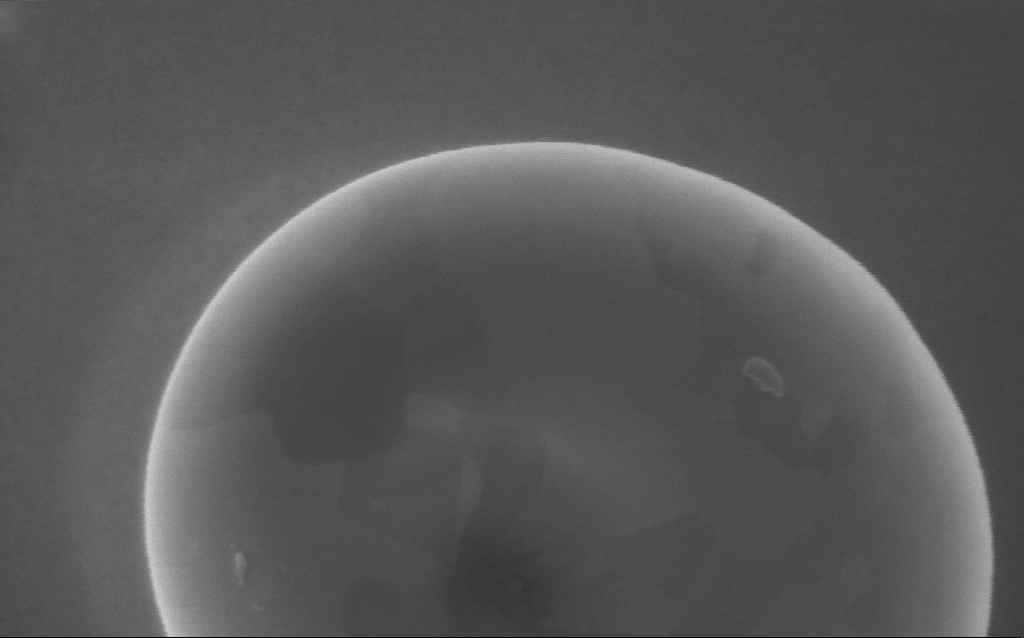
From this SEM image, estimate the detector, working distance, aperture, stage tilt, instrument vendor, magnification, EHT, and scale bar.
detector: InLens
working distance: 4 mm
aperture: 30 µm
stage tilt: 0°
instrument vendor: Zeiss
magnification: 180 K X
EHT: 5 kV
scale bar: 100 nm